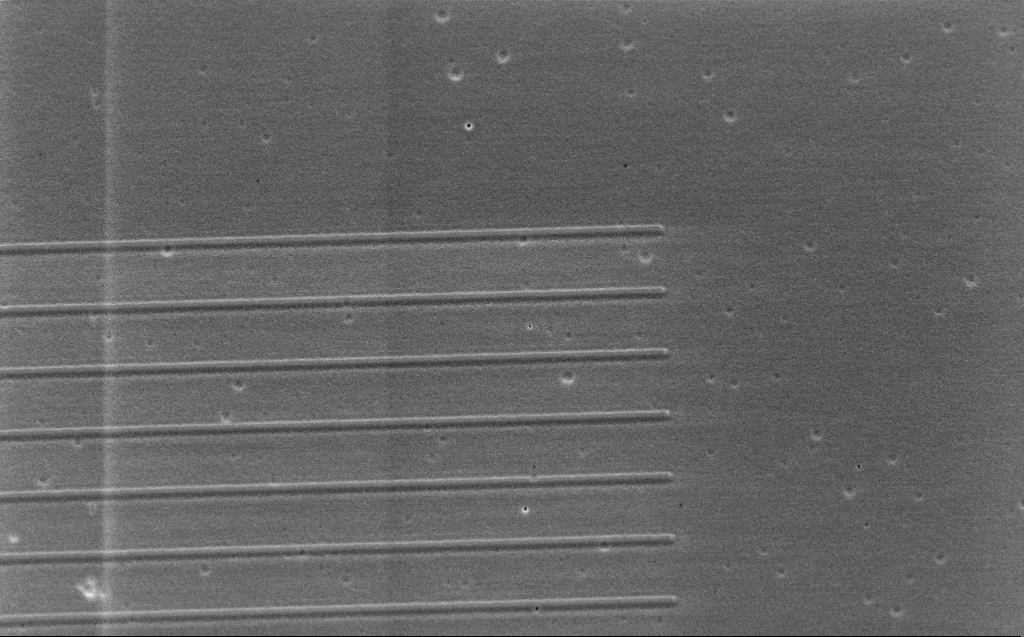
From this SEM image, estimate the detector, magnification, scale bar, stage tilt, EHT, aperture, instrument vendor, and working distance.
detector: InLens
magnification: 23.11 K X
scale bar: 2000 nm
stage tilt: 29.8°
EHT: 2 kV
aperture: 30 µm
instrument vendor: Zeiss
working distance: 4 mm